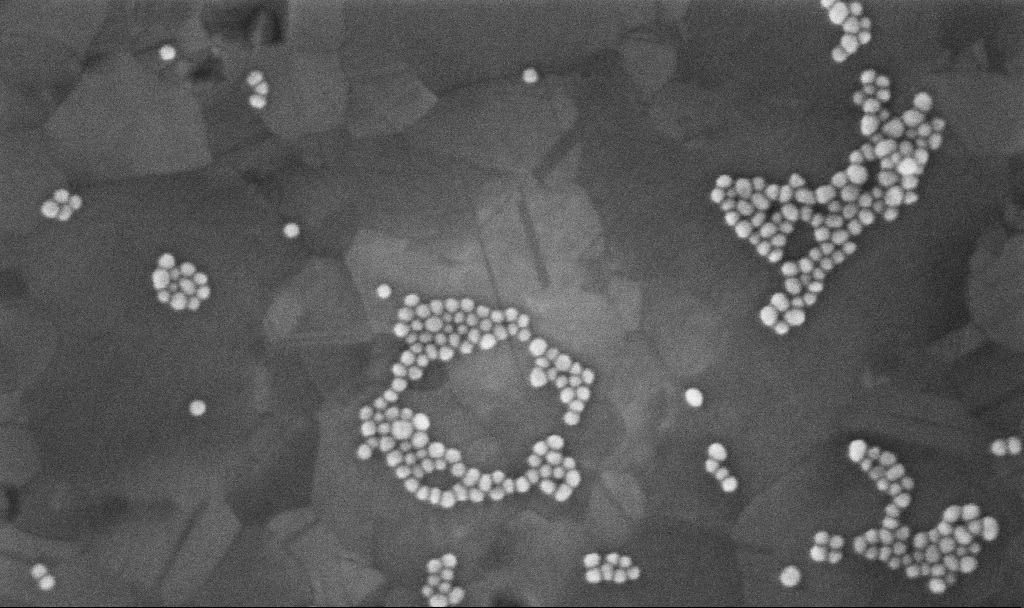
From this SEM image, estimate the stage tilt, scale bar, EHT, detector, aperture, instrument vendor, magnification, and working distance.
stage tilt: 0°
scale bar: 200 nm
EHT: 10 kV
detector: InLens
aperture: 30 µm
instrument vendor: Zeiss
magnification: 300 K X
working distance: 3.3 mm